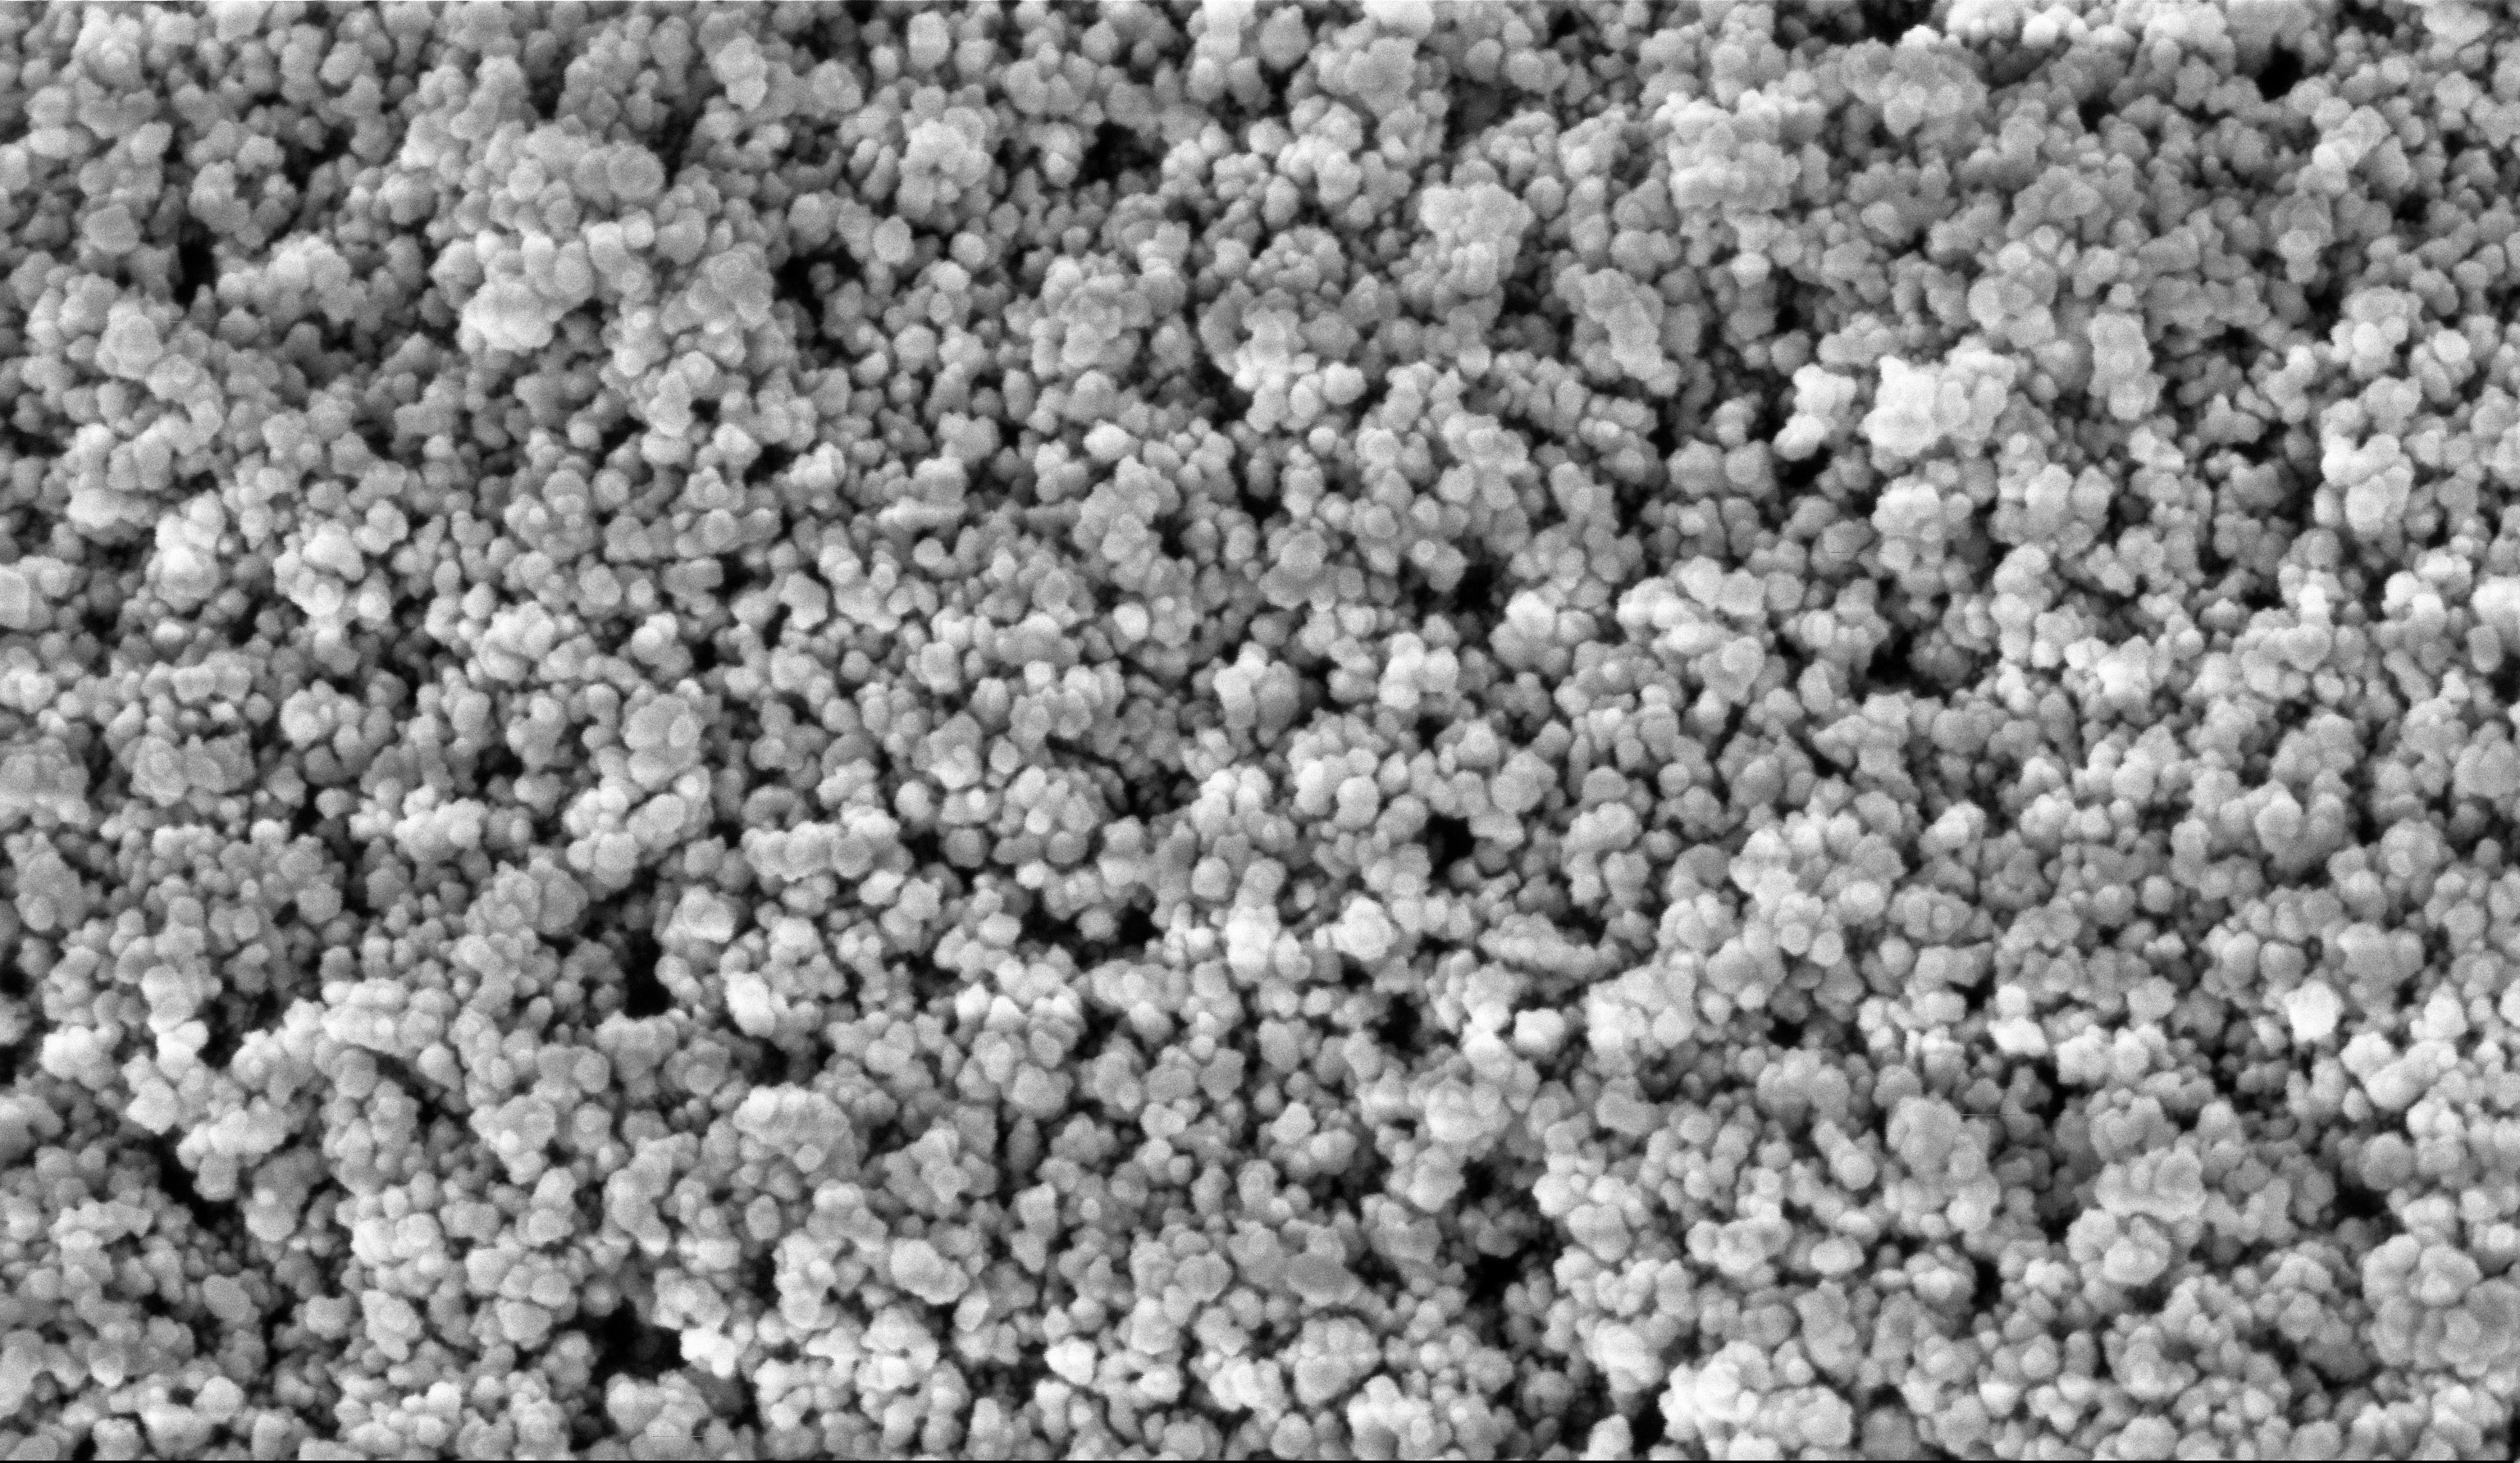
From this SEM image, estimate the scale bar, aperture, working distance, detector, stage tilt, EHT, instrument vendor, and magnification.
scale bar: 100 nm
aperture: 30 µm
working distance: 5.9 mm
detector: InLens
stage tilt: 0°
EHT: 5 kV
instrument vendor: Zeiss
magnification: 135 K X